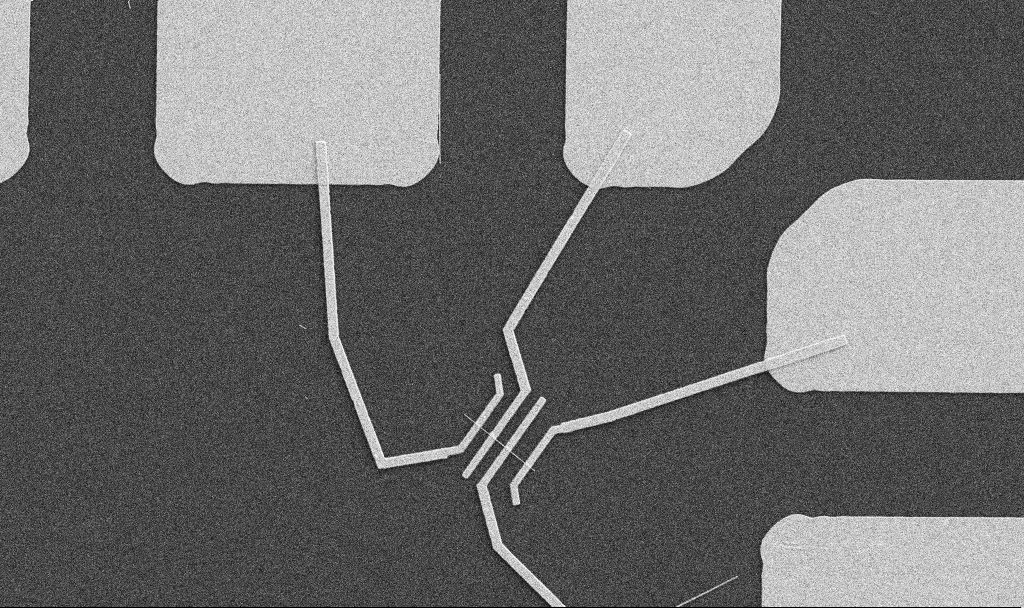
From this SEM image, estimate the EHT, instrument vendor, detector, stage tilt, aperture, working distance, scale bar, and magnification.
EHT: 5 kV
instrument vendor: Zeiss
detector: SE2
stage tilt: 0°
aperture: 30 µm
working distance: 10.7 mm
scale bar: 10000 nm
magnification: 5 K X